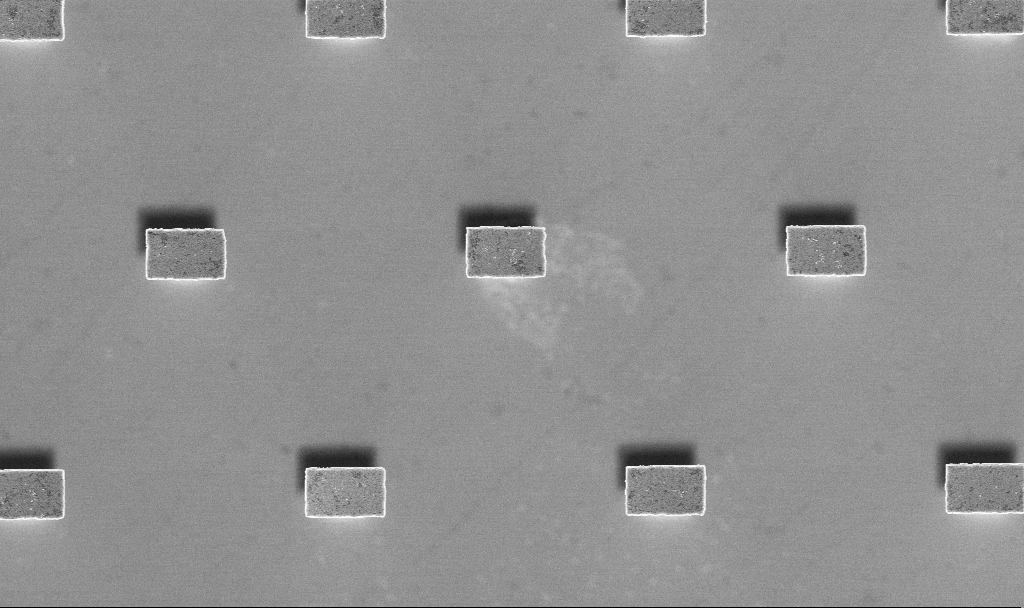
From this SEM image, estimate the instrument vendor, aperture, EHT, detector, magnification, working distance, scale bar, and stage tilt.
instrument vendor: Zeiss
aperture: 30 µm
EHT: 3 kV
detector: InLens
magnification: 9.97 K X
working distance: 3 mm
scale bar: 2000 nm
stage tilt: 0°